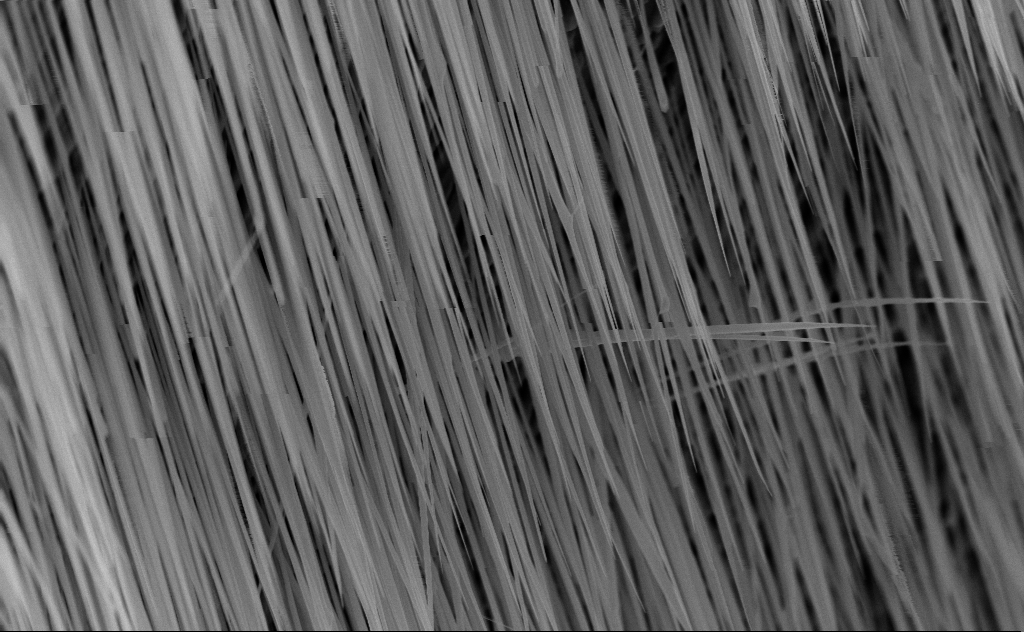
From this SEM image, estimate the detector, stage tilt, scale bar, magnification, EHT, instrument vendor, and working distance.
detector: InLens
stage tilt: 45°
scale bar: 2000 nm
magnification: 20 K X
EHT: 10 kV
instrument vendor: Zeiss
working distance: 6 mm